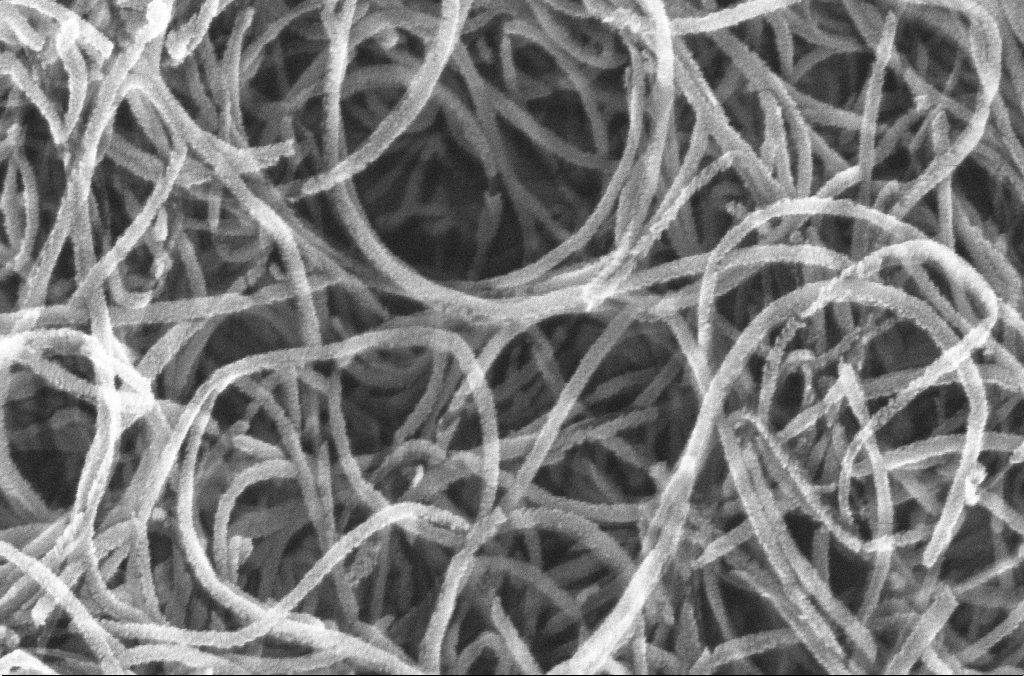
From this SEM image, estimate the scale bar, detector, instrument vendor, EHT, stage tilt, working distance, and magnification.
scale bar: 100 nm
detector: InLens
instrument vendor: Zeiss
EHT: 20 kV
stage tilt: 0°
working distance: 4.5 mm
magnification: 200 K X